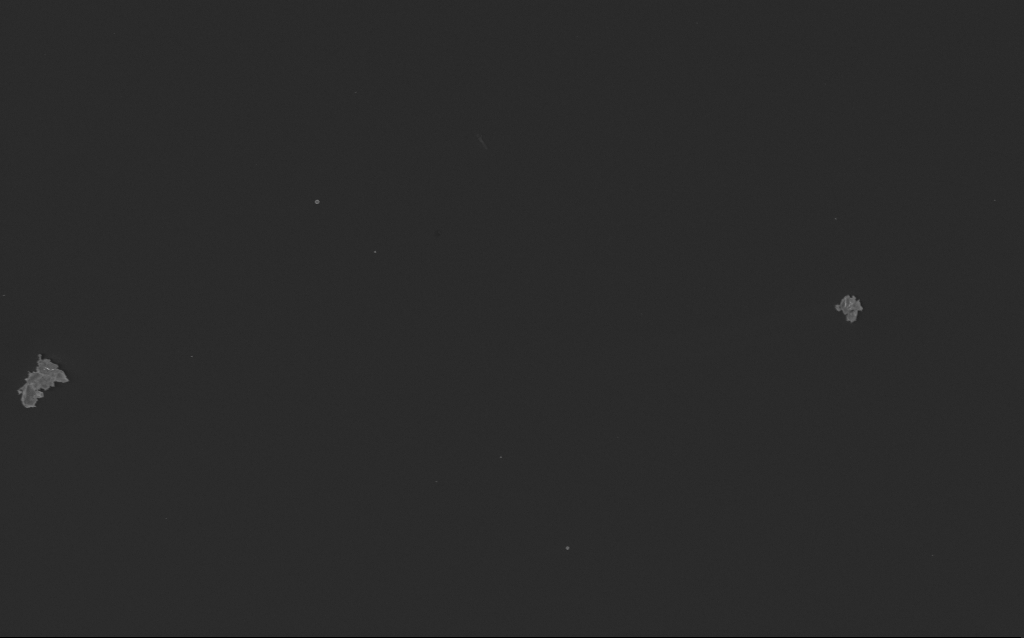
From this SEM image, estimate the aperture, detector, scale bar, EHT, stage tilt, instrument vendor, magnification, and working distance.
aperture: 30 µm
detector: InLens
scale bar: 10000 nm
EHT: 5 kV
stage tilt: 0°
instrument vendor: Zeiss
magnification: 5.89 K X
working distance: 3 mm